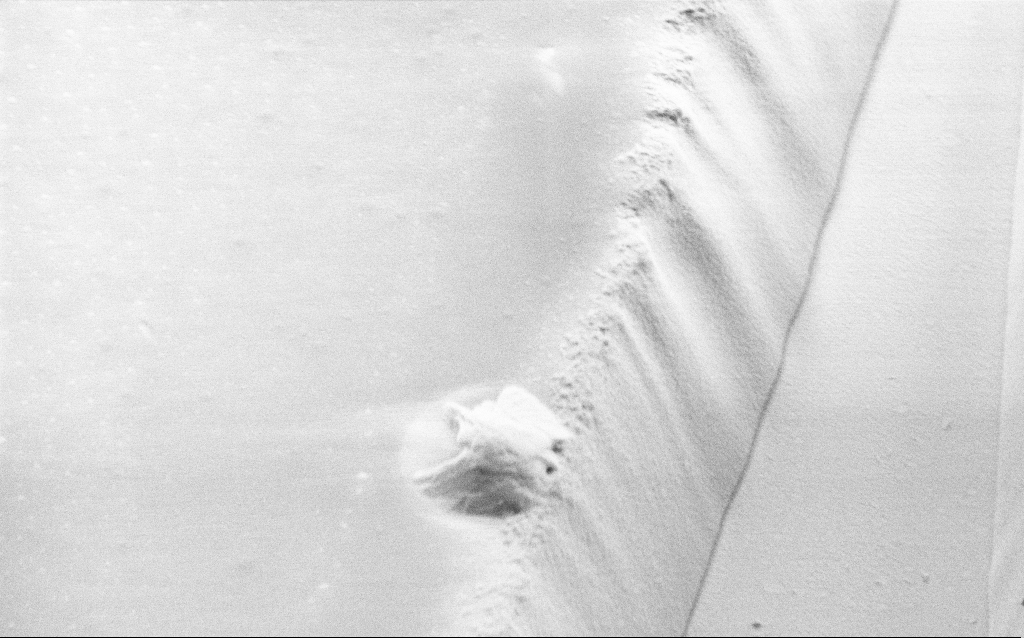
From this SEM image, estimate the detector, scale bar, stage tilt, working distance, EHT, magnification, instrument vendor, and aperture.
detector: SE2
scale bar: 2000 nm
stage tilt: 45°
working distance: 8 mm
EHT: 1.2 kV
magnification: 16.38 K X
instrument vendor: Zeiss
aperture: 30 µm